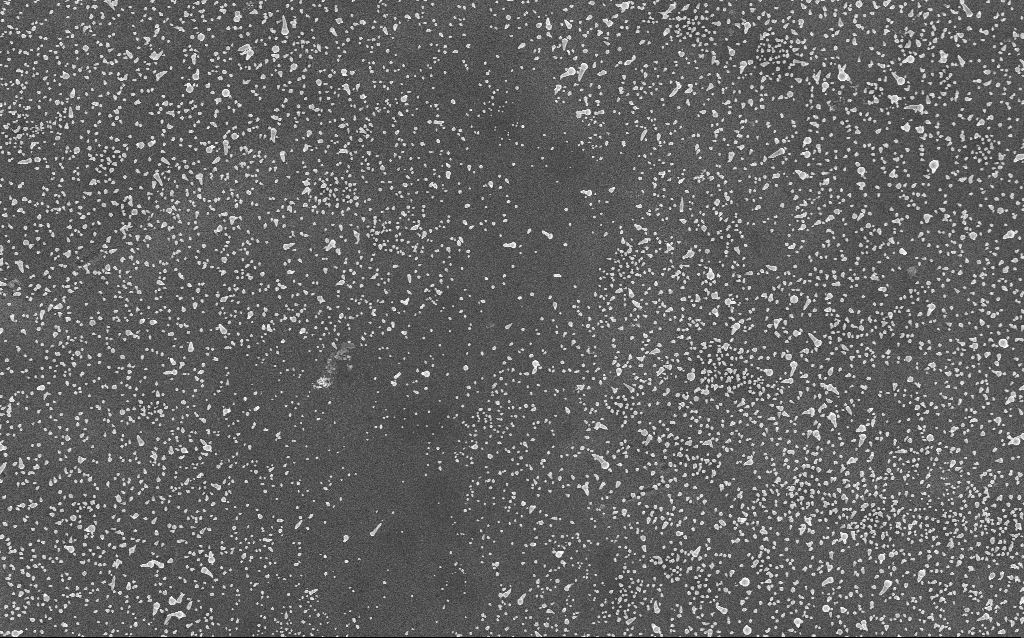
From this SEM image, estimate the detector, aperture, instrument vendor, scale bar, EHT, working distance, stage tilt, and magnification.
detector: InLens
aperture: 30 µm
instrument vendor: Zeiss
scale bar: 2000 nm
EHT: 5 kV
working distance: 4 mm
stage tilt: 0°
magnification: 10 K X